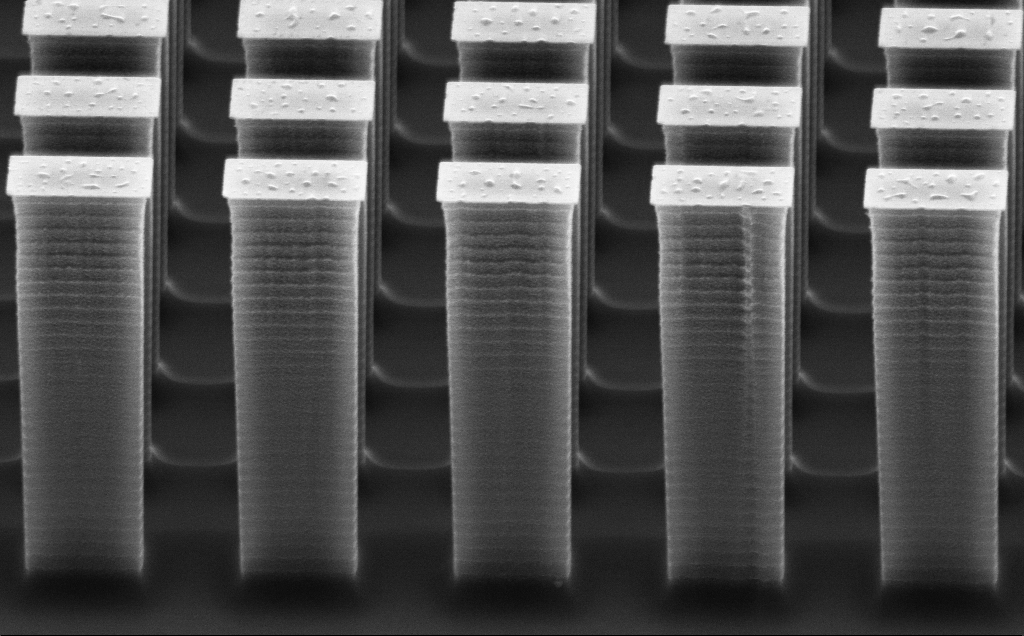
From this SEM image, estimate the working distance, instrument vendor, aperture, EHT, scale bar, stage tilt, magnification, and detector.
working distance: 15 mm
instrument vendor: Zeiss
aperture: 30 µm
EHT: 10 kV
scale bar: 2000 nm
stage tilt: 60.2°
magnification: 13.11 K X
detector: SE2